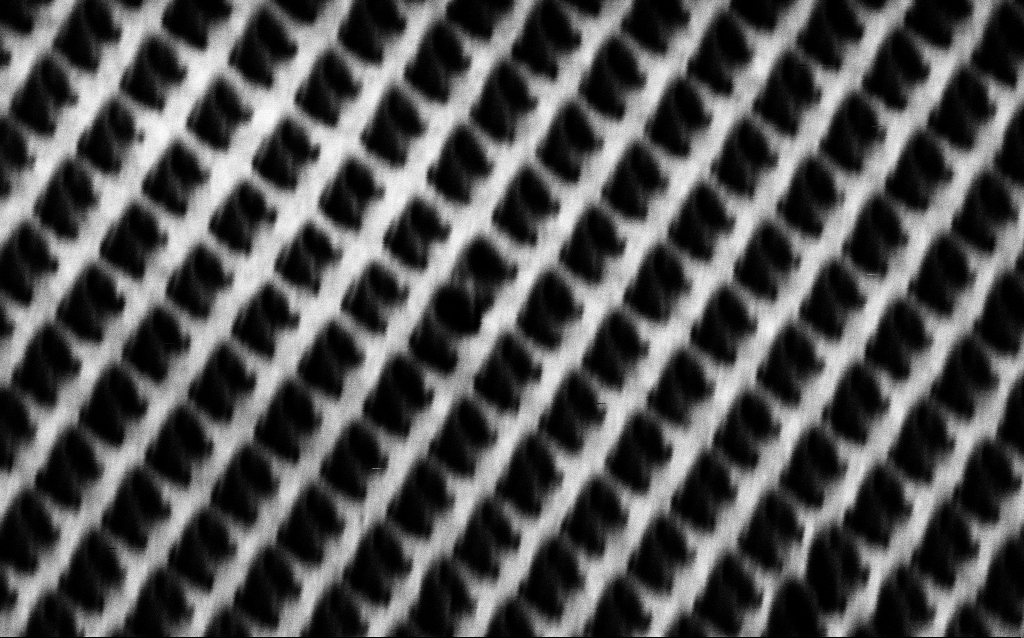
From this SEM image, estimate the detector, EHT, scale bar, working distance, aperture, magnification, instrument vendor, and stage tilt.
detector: SE2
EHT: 1.5 kV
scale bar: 1000 nm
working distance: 7.8 mm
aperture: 30 µm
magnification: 62.26 K X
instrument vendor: Zeiss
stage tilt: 45°